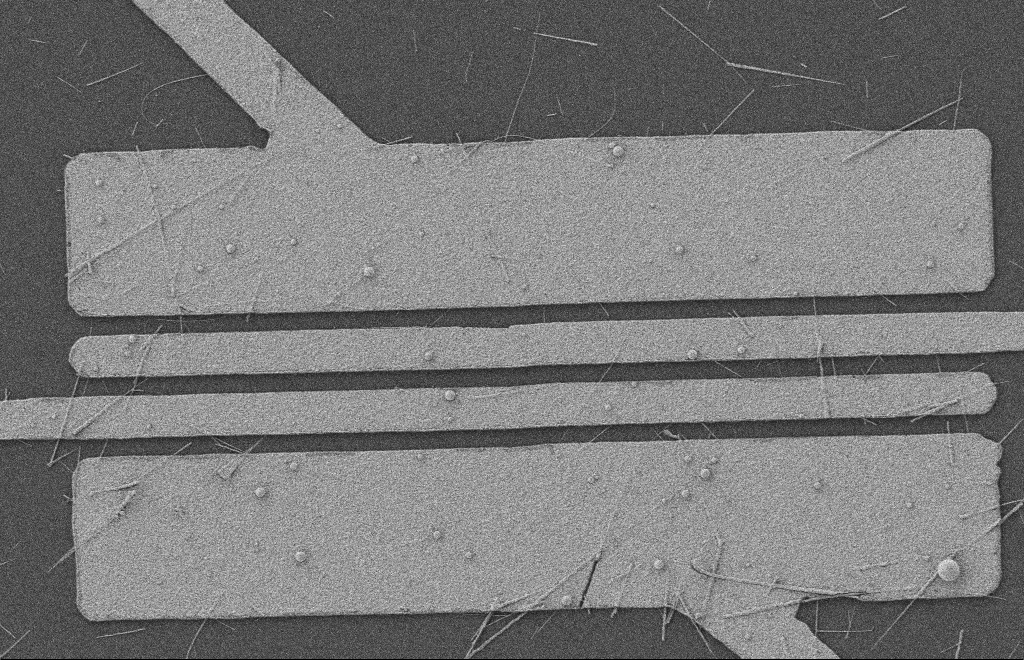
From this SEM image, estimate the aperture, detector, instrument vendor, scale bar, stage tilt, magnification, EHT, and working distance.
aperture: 20 µm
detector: SE2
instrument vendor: Zeiss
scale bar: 2000 nm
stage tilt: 0°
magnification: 5.58 K X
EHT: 2 kV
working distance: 8 mm